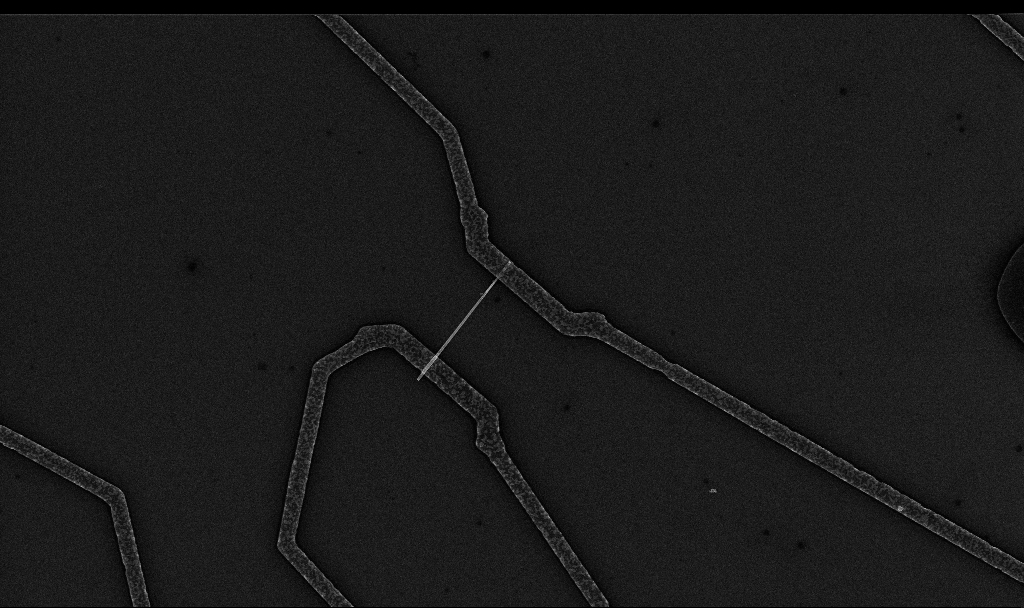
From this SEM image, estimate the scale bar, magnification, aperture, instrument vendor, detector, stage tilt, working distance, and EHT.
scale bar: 2000 nm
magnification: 8.98 K X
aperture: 30 µm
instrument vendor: Zeiss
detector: InLens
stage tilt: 0°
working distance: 6.7 mm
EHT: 10 kV